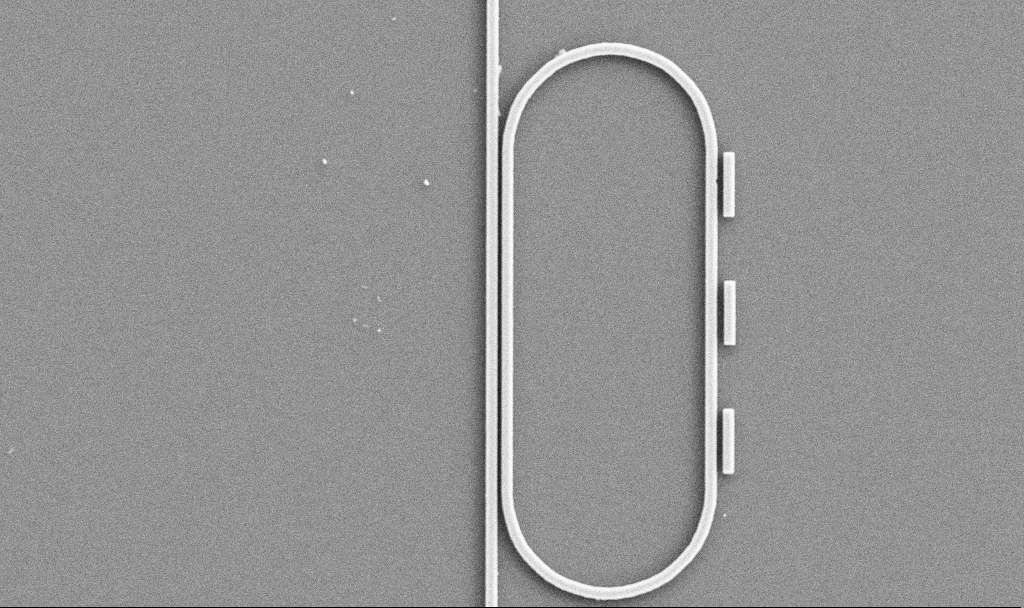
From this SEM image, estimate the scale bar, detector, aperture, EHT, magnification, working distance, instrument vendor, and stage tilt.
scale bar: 2000 nm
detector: SE2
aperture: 30 µm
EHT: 5 kV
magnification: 7.91 K X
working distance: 7.4 mm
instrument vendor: Zeiss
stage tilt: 0°